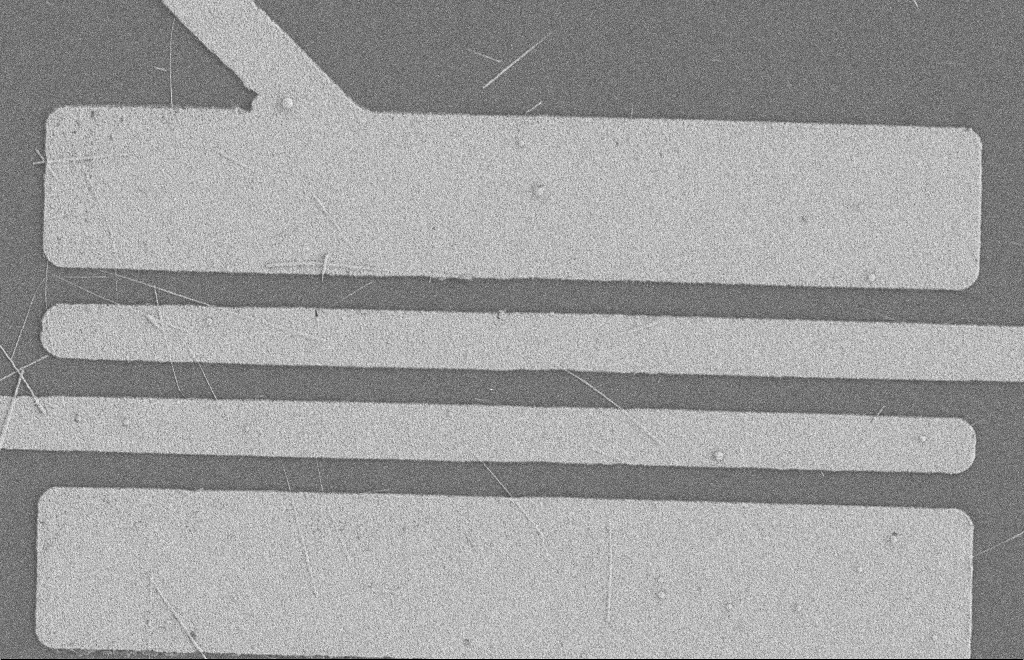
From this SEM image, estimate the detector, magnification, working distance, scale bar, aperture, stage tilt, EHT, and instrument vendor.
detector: SE2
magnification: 5.61 K X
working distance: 8 mm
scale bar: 2000 nm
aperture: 20 µm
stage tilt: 0°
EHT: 2 kV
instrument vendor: Zeiss